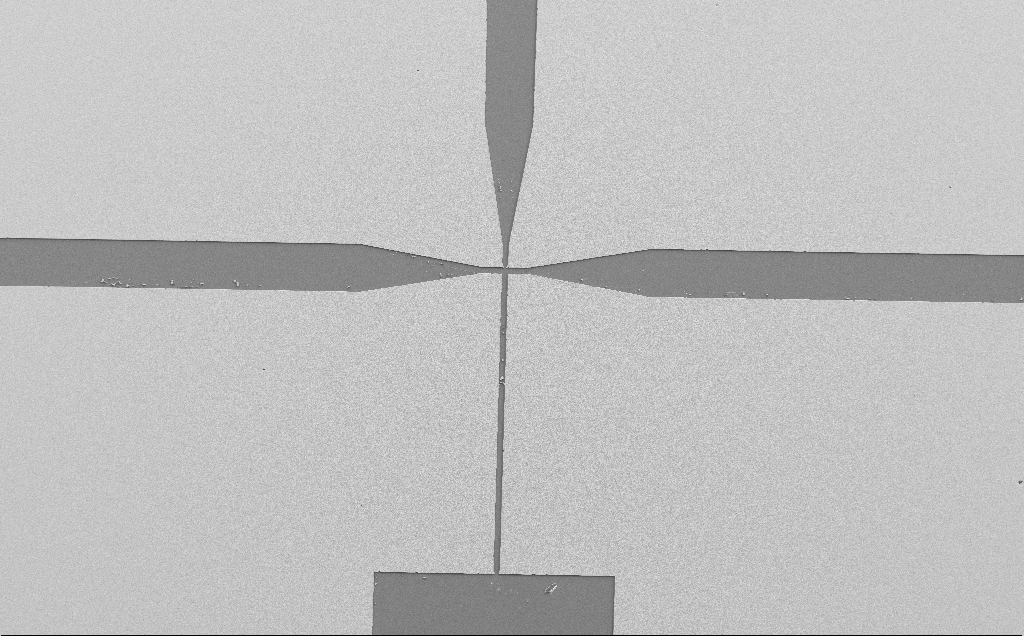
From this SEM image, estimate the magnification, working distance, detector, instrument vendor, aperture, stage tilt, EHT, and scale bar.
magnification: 0.223 K X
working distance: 9 mm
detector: SE2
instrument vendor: Zeiss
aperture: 30 µm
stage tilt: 0°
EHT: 10 kV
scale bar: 100000 nm